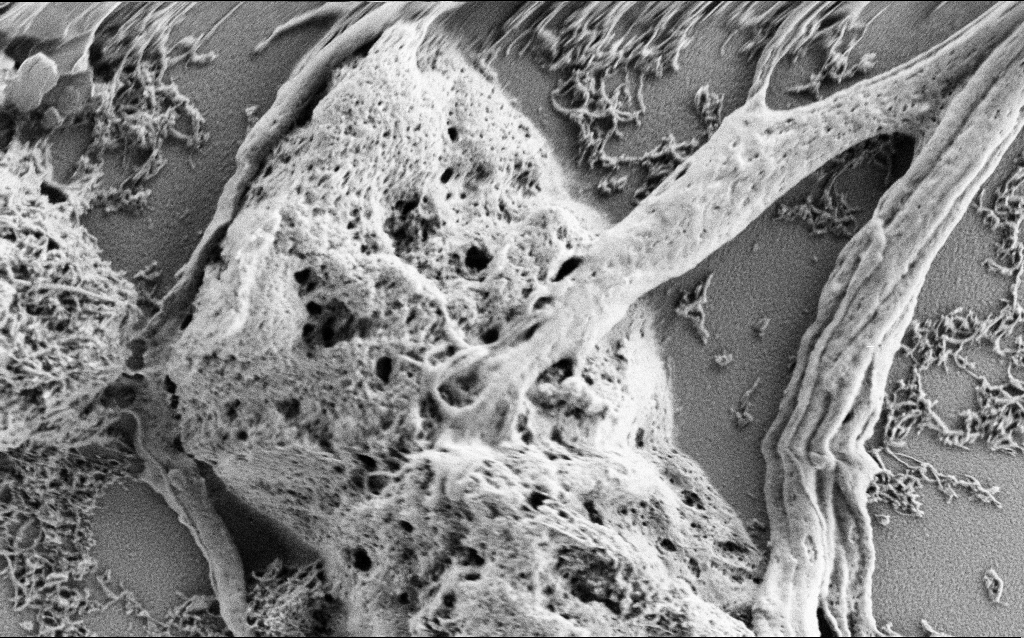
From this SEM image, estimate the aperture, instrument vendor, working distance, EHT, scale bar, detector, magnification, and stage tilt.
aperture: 30 µm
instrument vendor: Zeiss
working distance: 4 mm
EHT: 1 kV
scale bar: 1000 nm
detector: SE2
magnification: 25 K X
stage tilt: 0°